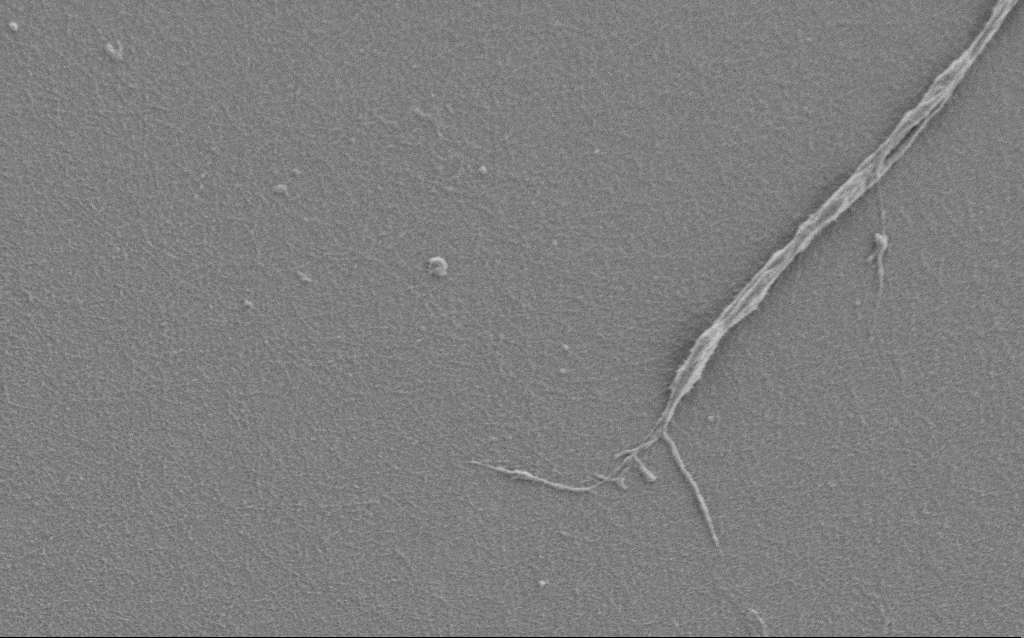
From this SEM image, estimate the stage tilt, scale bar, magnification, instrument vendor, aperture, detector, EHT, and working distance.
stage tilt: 0°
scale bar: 2000 nm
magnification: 7.5 K X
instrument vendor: Zeiss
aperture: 30 µm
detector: SE2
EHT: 1 kV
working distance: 6 mm